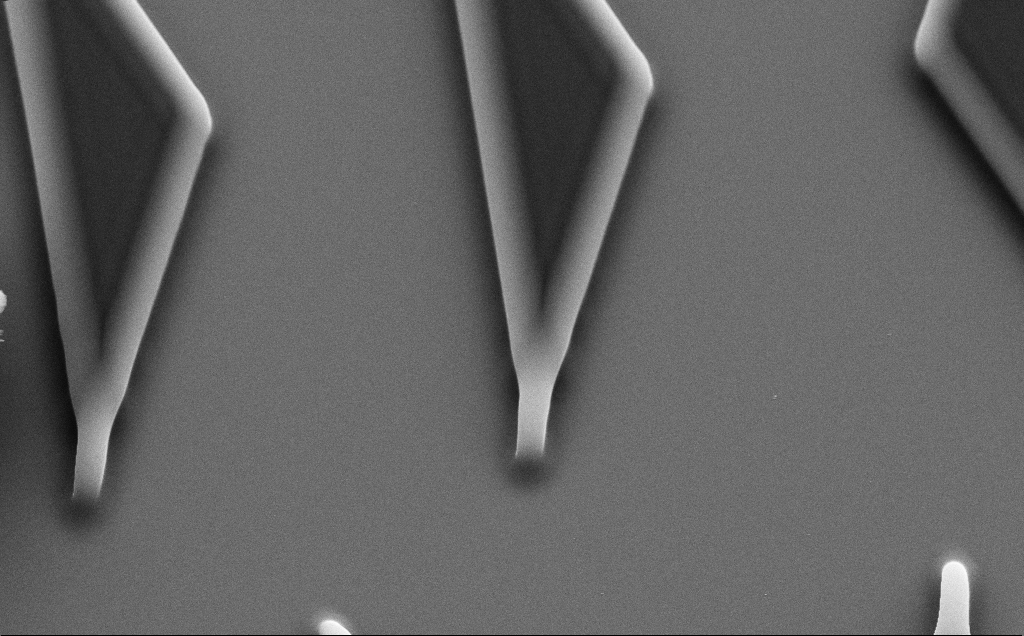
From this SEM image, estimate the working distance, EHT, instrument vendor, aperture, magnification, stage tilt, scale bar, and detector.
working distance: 8 mm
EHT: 10 kV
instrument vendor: Zeiss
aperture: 30 µm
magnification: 9.63 K X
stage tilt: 35°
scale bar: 2000 nm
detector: SE2